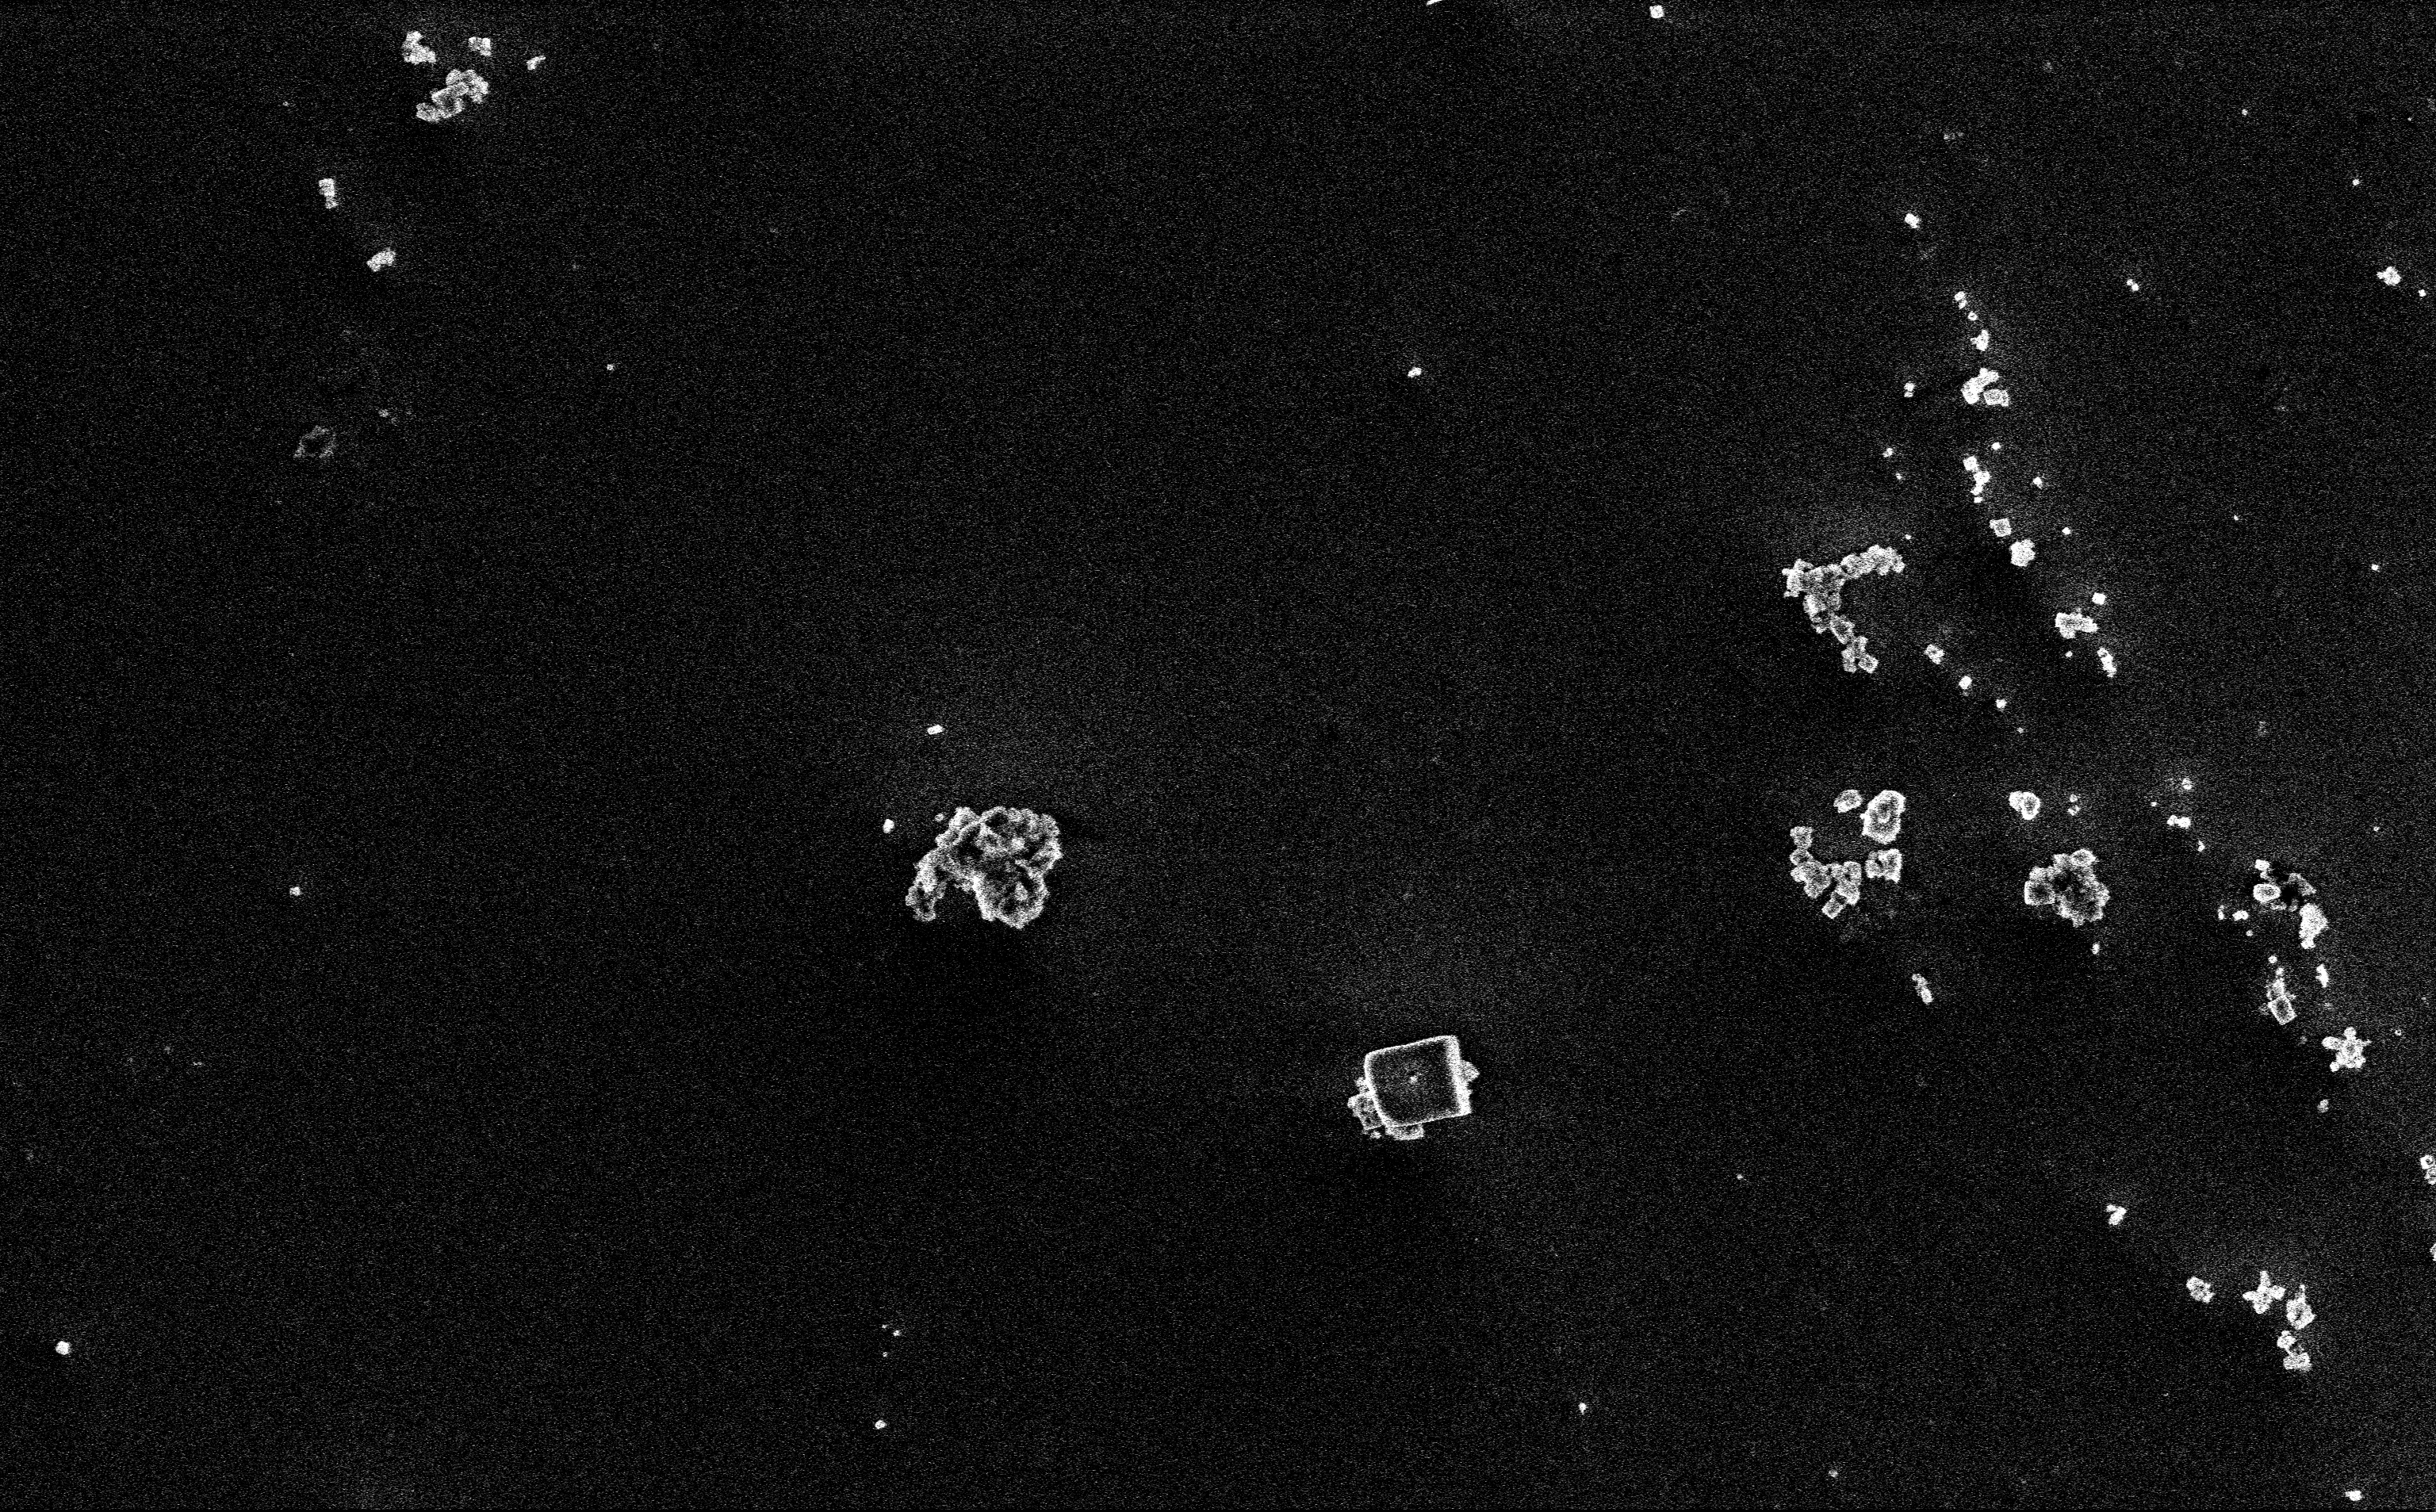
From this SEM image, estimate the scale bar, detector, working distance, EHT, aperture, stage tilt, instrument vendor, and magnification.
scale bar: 2000 nm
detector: InLens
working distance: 3 mm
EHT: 3 kV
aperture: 30 µm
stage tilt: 0°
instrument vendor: Zeiss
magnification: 12.85 K X